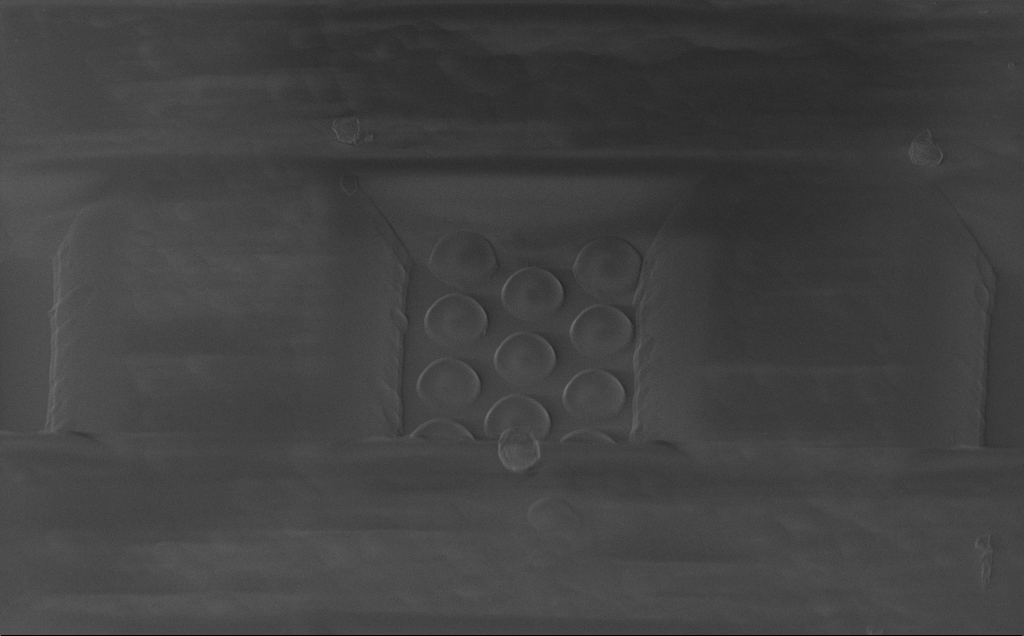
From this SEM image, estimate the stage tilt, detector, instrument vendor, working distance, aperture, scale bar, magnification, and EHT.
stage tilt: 38.2°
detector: InLens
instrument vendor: Zeiss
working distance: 6 mm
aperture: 30 µm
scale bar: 20000 nm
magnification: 1.49 K X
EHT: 1.3 kV